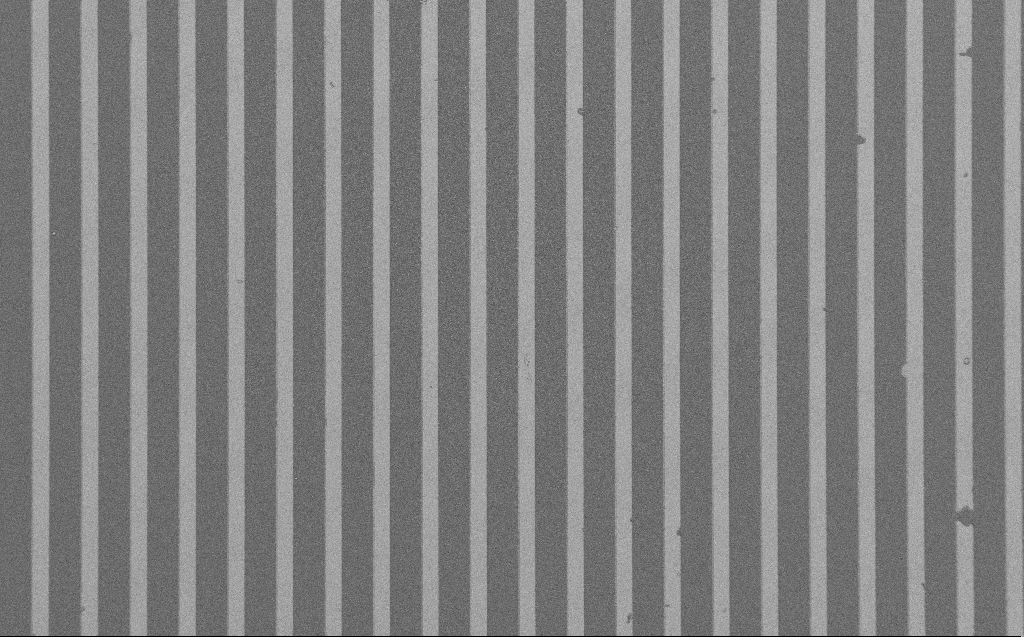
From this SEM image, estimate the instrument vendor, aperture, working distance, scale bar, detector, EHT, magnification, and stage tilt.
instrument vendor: Zeiss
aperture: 30 µm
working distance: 4 mm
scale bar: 100000 nm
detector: SE2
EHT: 1.2 kV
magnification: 0.649 K X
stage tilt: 0°